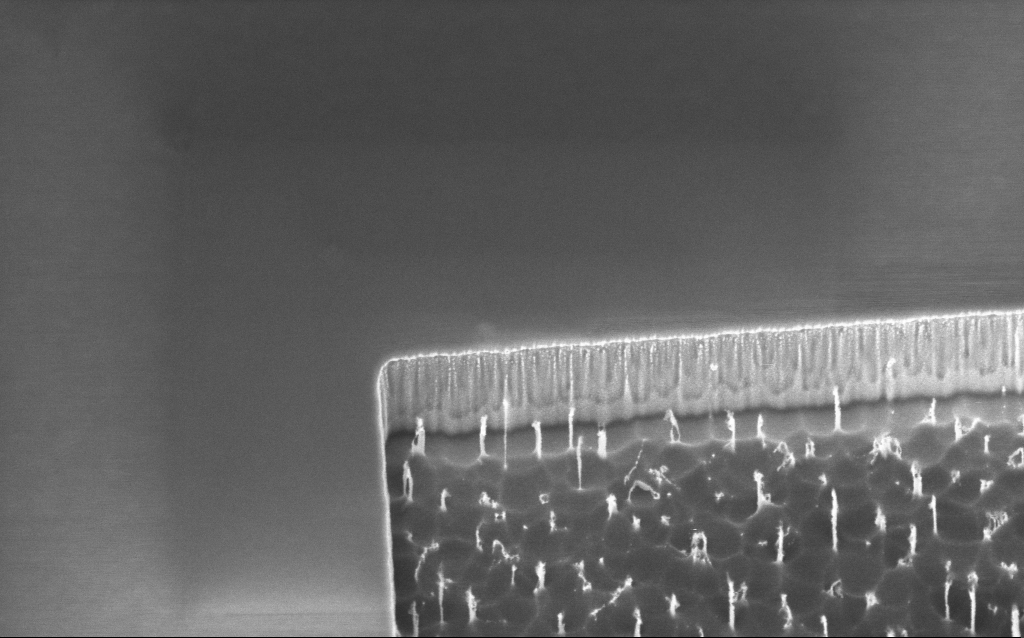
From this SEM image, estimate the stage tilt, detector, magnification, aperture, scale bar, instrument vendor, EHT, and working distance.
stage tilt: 45°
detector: InLens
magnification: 47.54 K X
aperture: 30 µm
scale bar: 1000 nm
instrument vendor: Zeiss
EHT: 2 kV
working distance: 6 mm